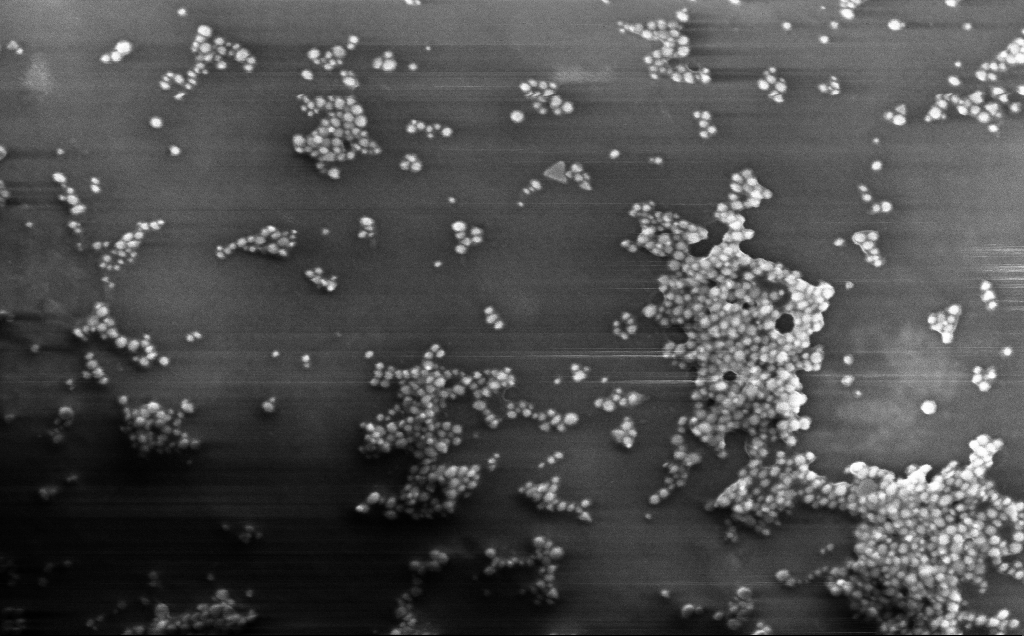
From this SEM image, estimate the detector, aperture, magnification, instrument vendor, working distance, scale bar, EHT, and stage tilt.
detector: InLens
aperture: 30 µm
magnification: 159.96 K X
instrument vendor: Zeiss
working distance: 4 mm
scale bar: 200 nm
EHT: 10 kV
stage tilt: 0°